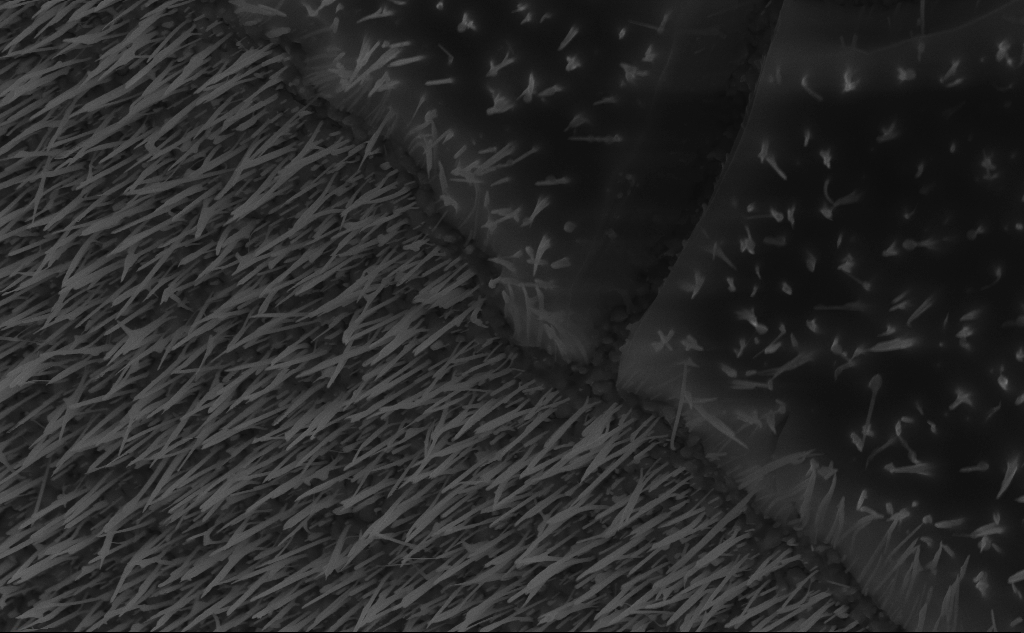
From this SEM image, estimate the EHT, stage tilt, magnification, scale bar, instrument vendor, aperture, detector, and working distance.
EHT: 10 kV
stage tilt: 45°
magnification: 30.35 K X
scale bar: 2000 nm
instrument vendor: Zeiss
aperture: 30 µm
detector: InLens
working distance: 6 mm